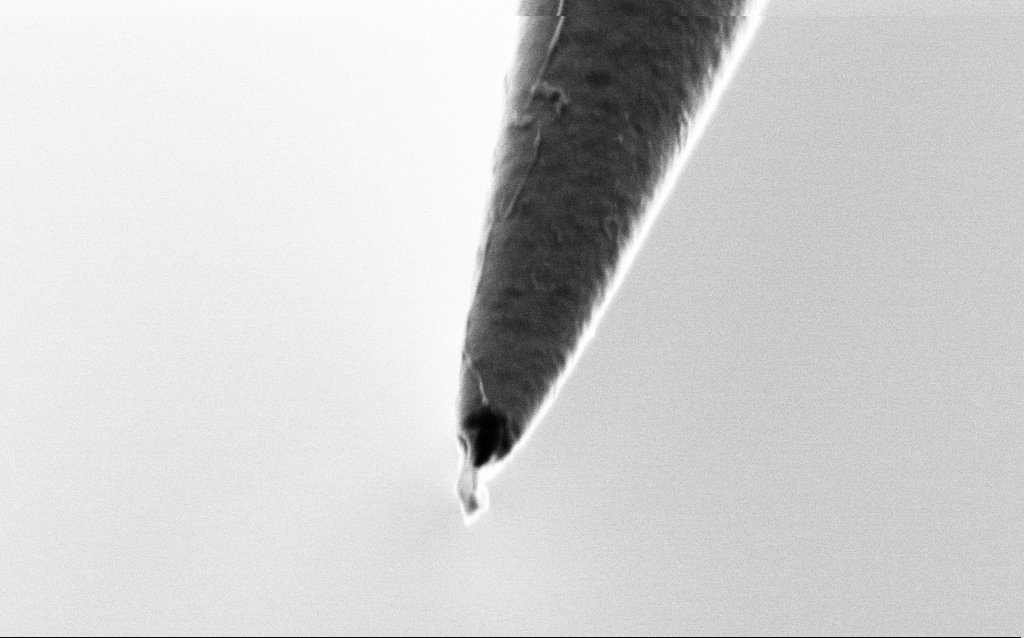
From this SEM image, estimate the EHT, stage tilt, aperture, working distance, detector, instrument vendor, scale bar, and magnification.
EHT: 1 kV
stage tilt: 45°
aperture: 30 µm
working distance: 6 mm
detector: SE2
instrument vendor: Zeiss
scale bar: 200 nm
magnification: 75 K X